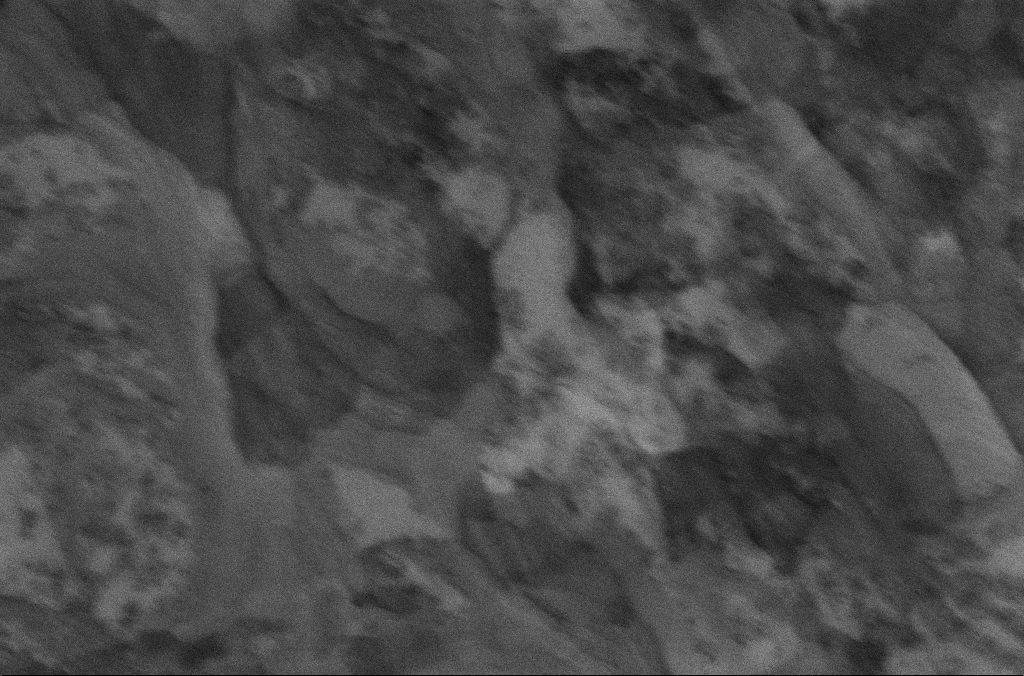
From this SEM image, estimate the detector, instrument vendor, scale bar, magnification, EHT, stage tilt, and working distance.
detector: InLens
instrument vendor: Zeiss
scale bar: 200 nm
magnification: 100 K X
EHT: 5 kV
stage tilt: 0°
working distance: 6.7 mm